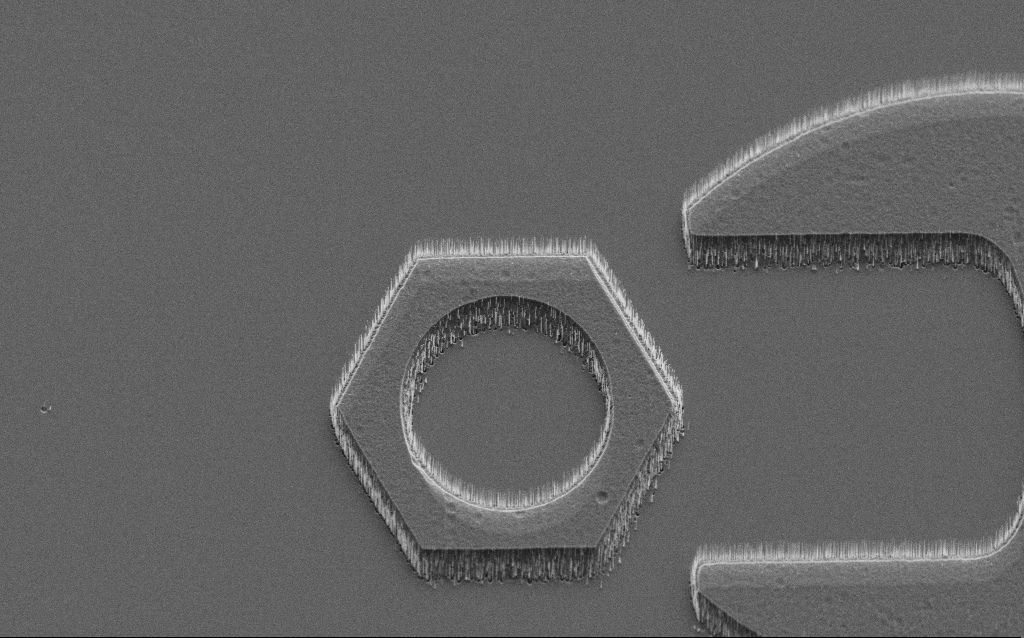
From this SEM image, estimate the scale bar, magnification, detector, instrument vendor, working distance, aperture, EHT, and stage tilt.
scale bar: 20000 nm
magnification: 2.3 K X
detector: SE2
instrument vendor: Zeiss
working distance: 8 mm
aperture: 30 µm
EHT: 5 kV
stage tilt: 45°